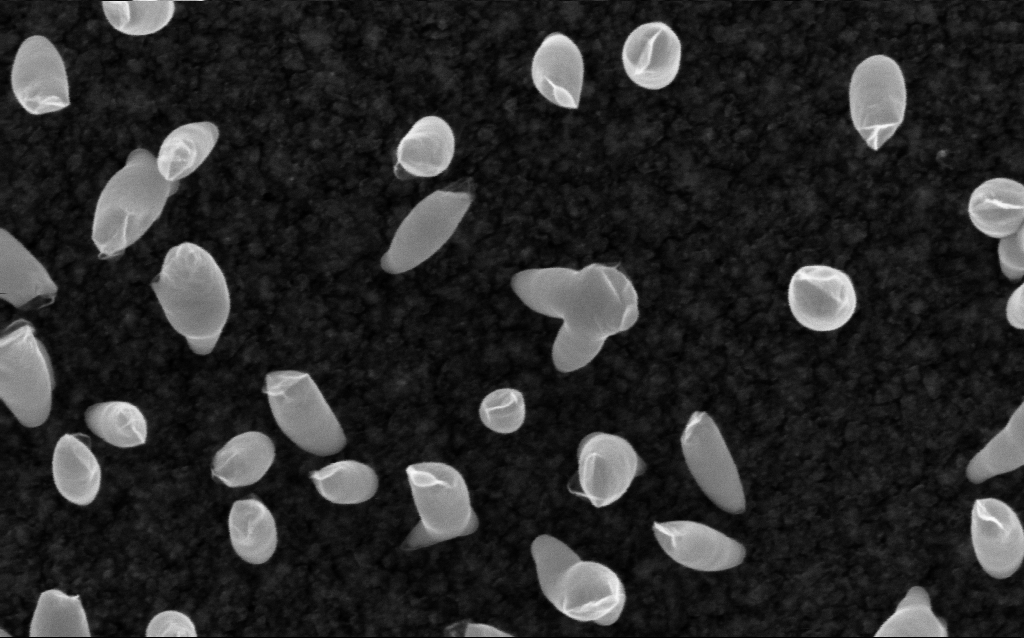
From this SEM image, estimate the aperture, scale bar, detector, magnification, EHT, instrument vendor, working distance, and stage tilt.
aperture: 30 µm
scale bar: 100 nm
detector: InLens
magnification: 200 K X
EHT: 5 kV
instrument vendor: Zeiss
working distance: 2.9 mm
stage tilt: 0°